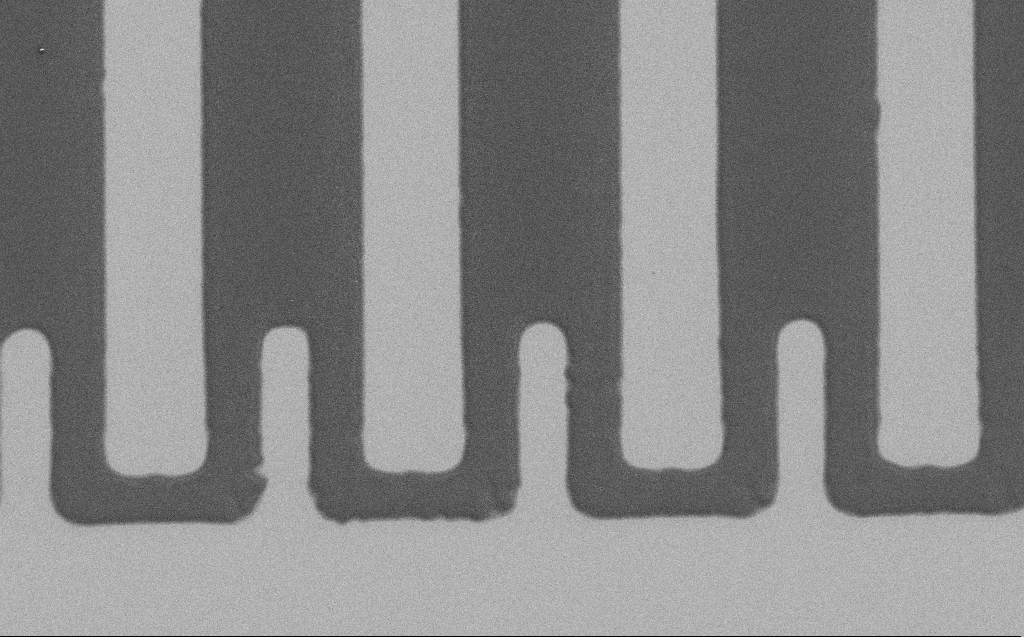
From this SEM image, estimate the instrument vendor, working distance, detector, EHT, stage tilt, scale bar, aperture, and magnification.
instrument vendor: Zeiss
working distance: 6 mm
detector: SE2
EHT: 1.2 kV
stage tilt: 0°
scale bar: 10000 nm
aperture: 30 µm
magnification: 3.42 K X